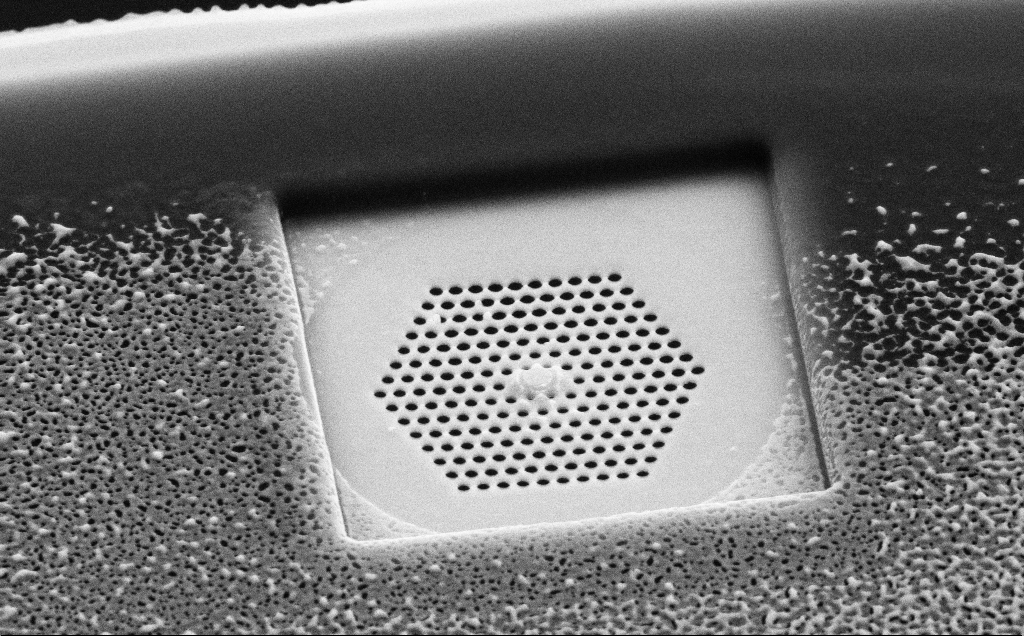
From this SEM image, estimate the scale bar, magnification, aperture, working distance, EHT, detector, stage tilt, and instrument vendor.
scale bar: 1000 nm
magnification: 29.59 K X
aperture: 30 µm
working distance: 8 mm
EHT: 10 kV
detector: SE2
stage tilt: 45°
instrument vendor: Zeiss